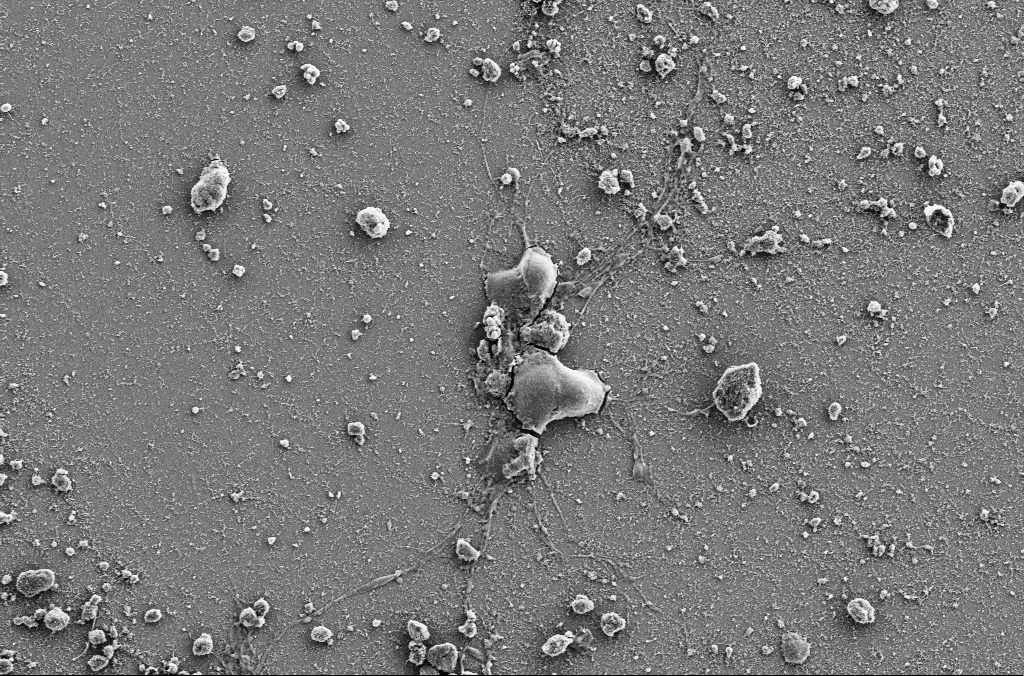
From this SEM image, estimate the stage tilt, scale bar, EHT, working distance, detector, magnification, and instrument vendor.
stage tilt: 0°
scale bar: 20000 nm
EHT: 5 kV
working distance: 4 mm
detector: SE2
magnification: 2.5 K X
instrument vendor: Zeiss